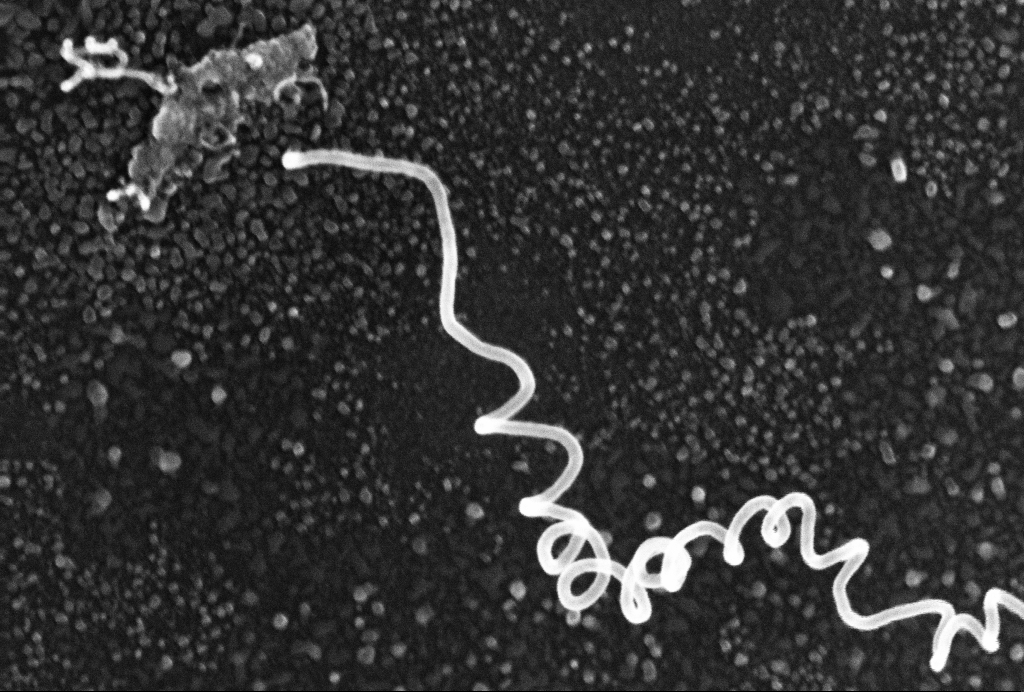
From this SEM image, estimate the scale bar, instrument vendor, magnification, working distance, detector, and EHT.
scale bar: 300 nm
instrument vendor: Zeiss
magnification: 126.67 K X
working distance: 3 mm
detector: InLens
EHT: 10 kV